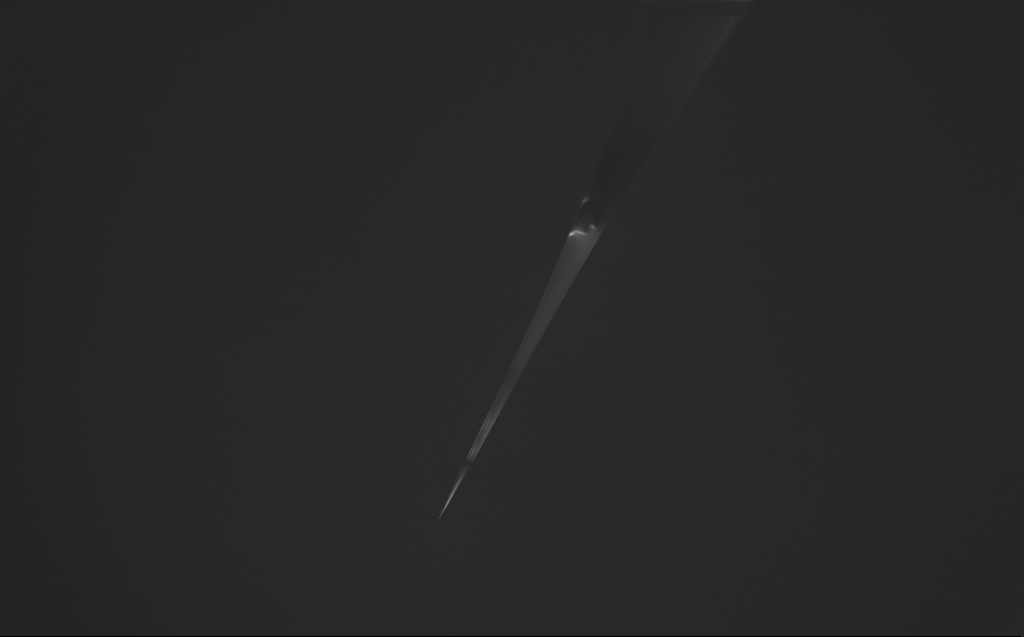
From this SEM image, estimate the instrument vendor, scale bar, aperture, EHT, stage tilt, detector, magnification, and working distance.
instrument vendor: Zeiss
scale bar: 200000 nm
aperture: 30 µm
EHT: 2 kV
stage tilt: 45°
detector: InLens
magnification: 0.1 K X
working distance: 6 mm